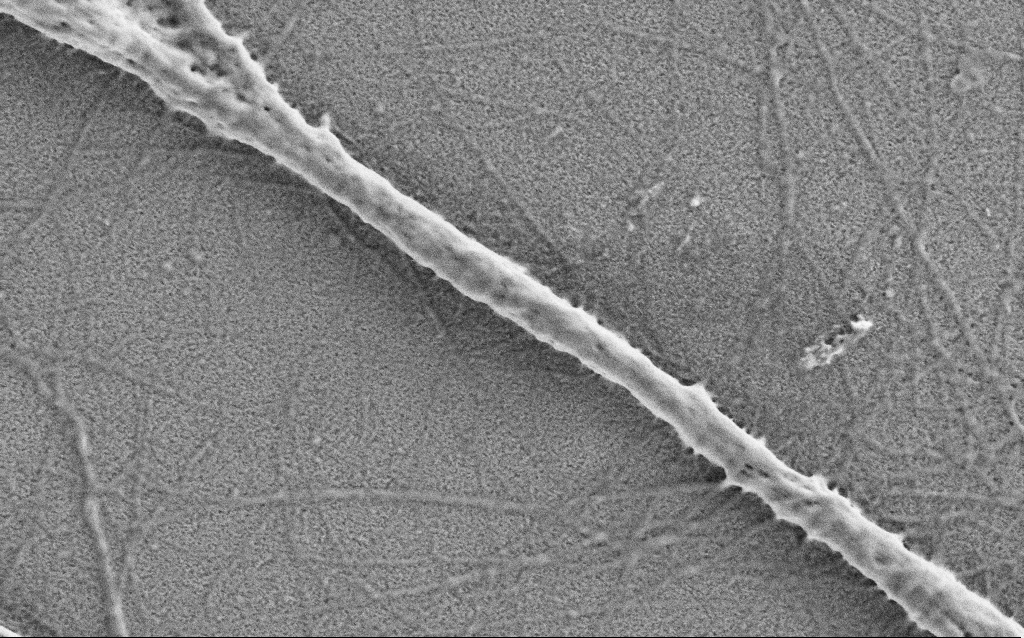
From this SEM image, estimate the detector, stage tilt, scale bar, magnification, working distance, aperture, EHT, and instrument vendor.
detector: SE2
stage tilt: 0°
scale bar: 1000 nm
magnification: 50 K X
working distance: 4 mm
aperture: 30 µm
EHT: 1 kV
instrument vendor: Zeiss